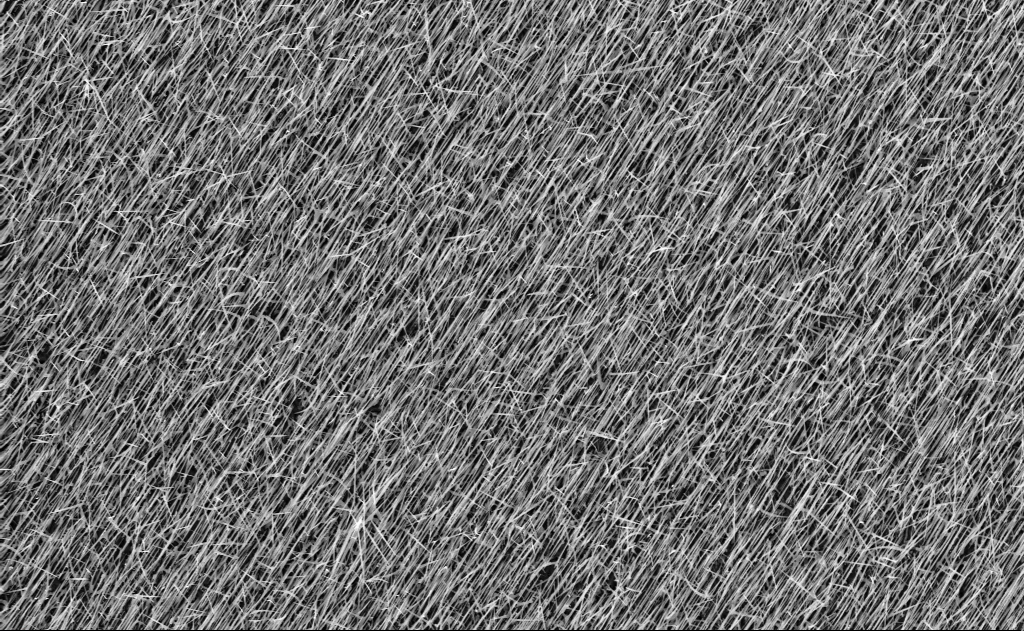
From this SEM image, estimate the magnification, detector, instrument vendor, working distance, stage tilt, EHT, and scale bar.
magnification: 5 K X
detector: InLens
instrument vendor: Zeiss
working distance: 7 mm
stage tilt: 0°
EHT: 10 kV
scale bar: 10000 nm